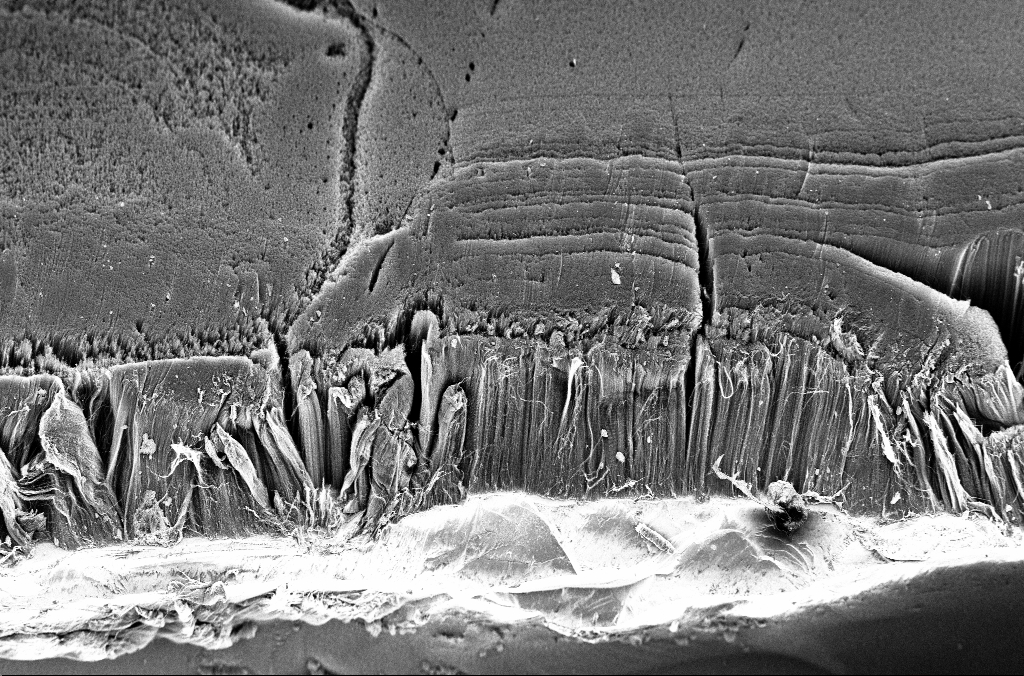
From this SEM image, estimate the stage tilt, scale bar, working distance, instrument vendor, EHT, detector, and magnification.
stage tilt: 45°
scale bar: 100000 nm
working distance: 3.3 mm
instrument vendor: Zeiss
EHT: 3 kV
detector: InLens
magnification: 0.5 K X